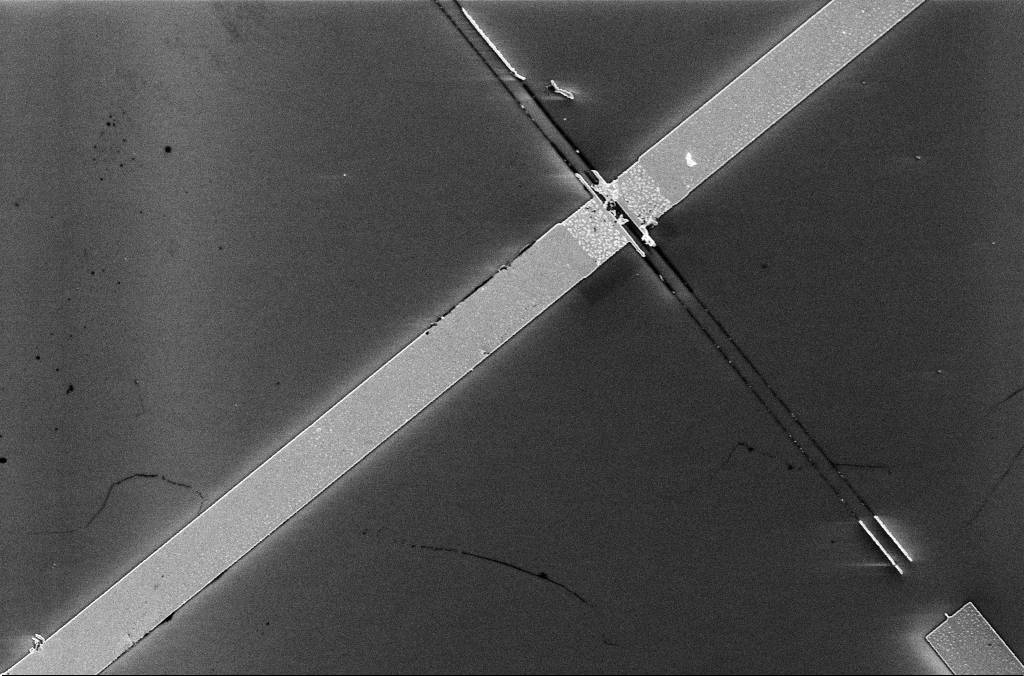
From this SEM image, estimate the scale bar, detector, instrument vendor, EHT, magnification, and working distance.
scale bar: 10000 nm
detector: InLens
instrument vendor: Zeiss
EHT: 5 kV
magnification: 4.28 K X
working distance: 3.3 mm